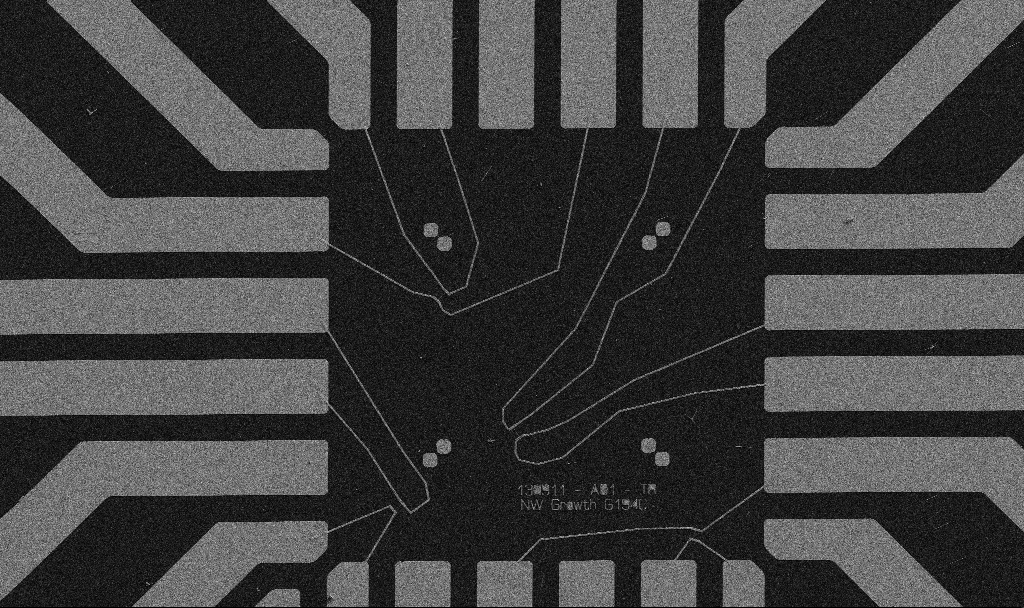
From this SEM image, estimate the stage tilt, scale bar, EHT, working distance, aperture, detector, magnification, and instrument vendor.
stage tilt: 0°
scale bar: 20000 nm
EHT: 5 kV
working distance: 10.7 mm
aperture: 30 µm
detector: SE2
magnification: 1 K X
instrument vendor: Zeiss